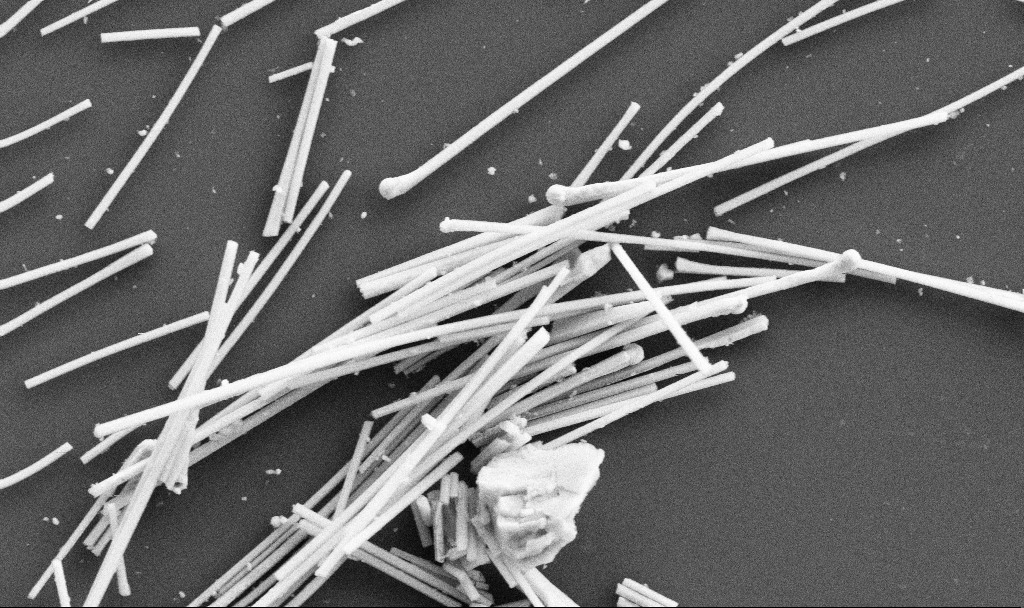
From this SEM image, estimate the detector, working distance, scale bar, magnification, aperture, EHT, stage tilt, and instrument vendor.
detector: SE2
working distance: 6.7 mm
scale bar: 1000 nm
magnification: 36.3 K X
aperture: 30 µm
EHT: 5 kV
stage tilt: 0°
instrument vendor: Zeiss